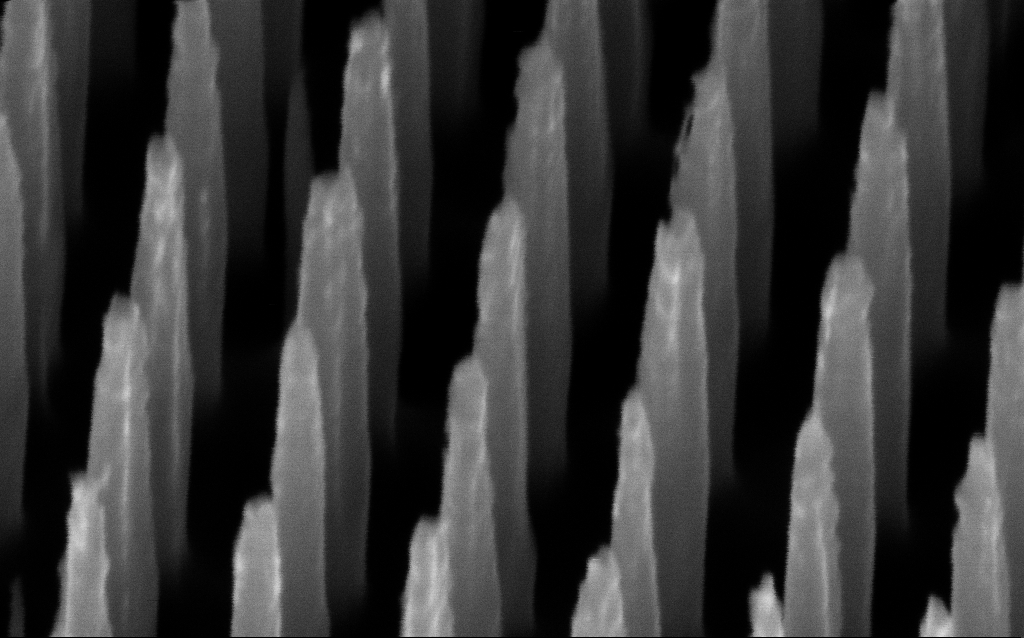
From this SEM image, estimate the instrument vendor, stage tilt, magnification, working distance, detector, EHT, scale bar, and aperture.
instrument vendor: Zeiss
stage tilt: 45°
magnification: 323.68 K X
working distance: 5 mm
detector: InLens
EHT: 3 kV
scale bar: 100 nm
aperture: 30 µm